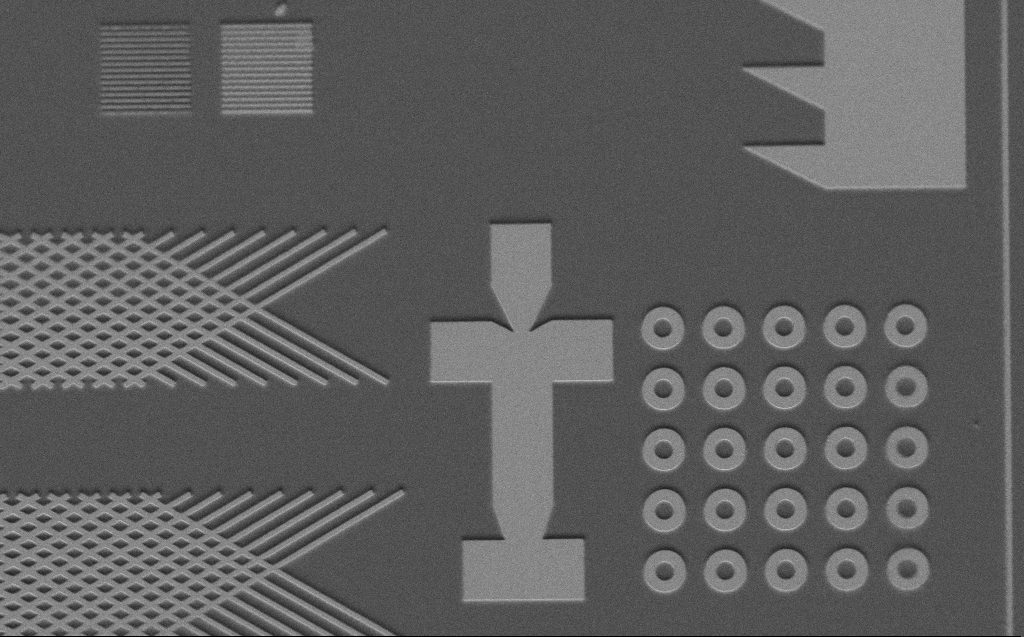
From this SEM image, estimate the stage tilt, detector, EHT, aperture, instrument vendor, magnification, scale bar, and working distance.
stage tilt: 45°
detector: SE2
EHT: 3 kV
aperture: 30 µm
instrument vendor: Zeiss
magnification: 2.24 K X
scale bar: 10000 nm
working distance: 6 mm